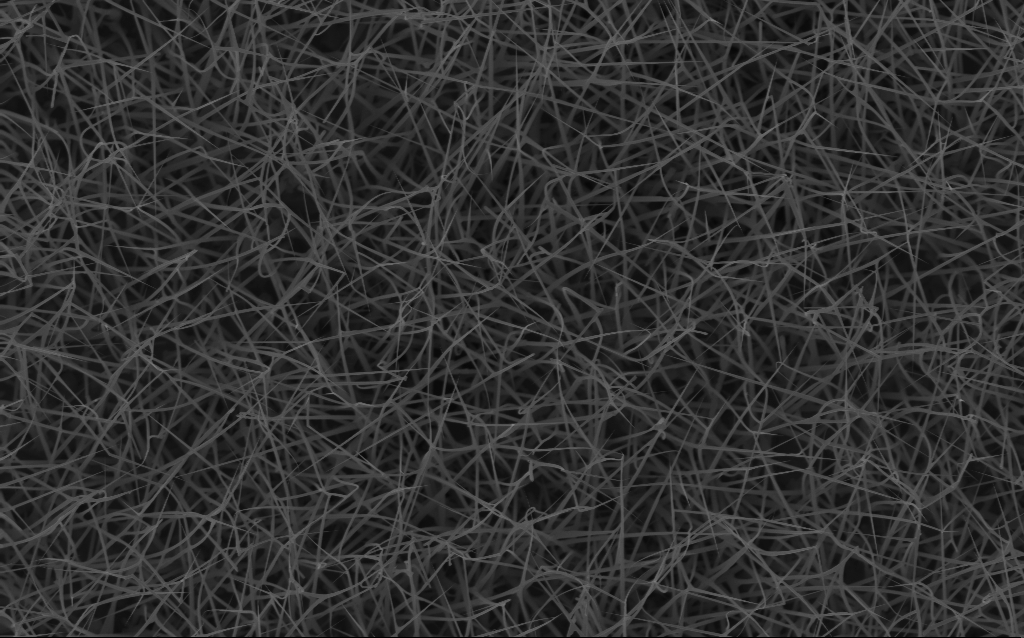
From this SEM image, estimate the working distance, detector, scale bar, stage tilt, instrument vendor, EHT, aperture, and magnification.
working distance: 6 mm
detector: InLens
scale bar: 2000 nm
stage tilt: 0°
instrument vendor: Zeiss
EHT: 10 kV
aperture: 30 µm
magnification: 10 K X